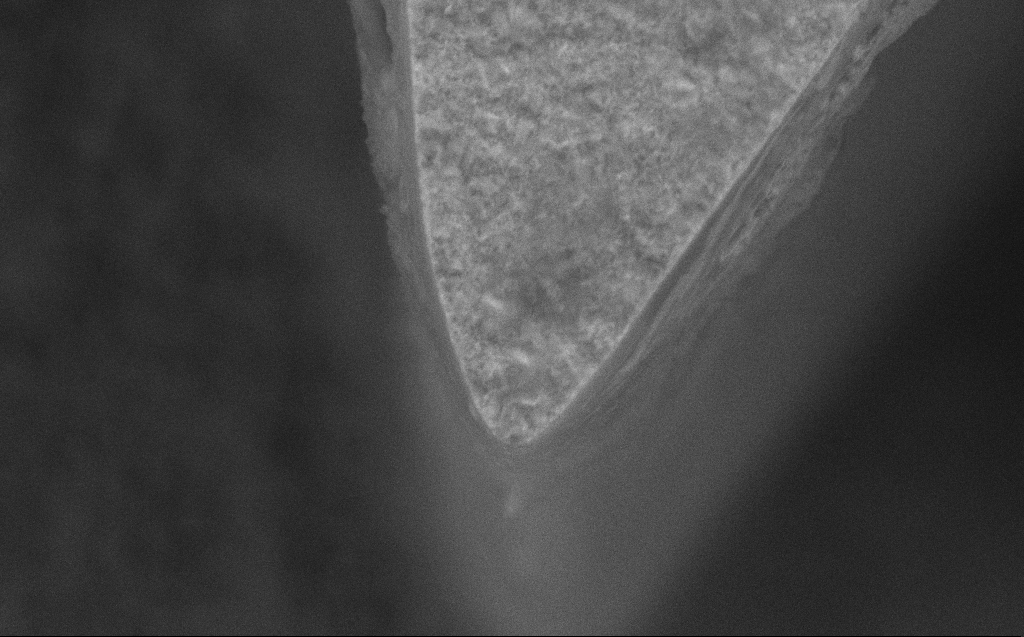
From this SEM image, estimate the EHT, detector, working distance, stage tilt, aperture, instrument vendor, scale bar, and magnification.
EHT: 5 kV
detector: SE2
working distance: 7 mm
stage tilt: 0°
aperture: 30 µm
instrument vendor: Zeiss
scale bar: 2000 nm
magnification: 33.29 K X